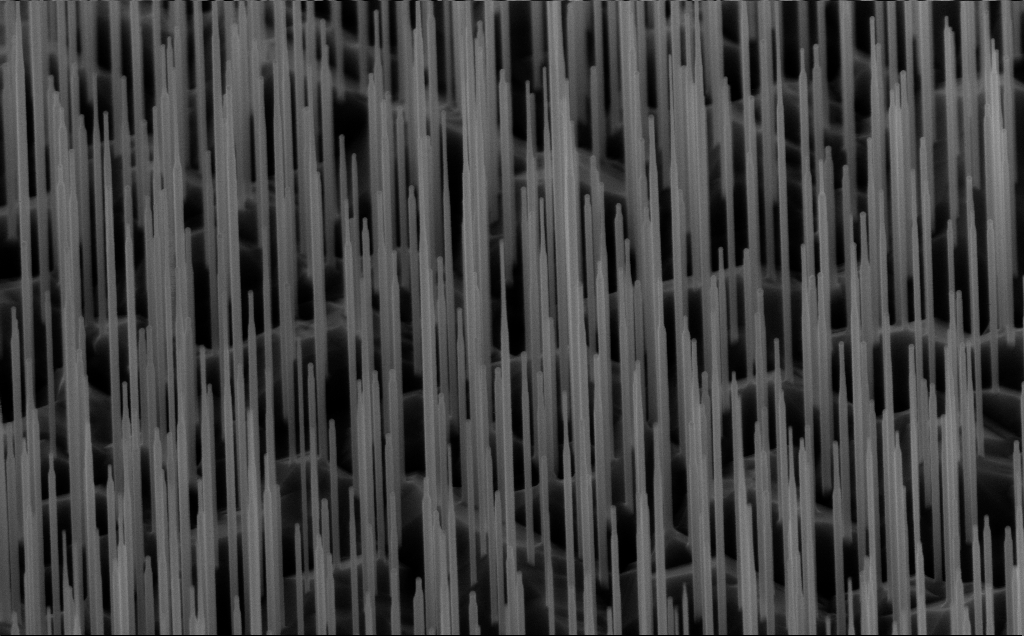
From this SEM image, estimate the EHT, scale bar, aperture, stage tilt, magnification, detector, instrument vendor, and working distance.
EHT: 10 kV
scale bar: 1000 nm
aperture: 30 µm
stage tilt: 45°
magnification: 40 K X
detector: InLens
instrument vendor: Zeiss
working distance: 6 mm